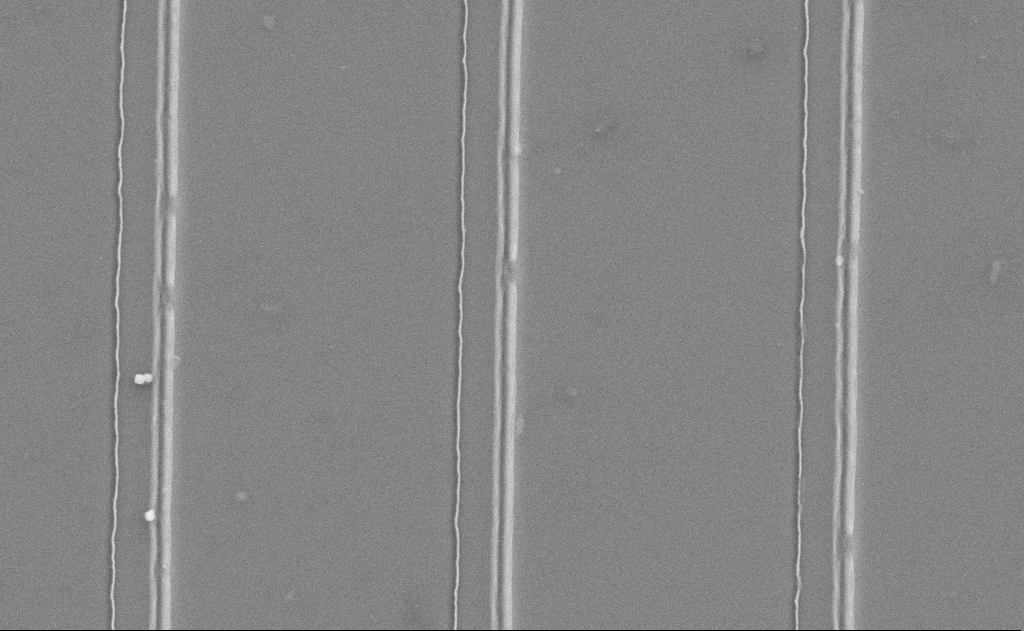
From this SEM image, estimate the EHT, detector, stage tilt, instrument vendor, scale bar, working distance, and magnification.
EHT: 5 kV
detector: SE2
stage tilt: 0°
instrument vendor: Zeiss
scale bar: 2000 nm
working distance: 12 mm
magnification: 31.17 K X